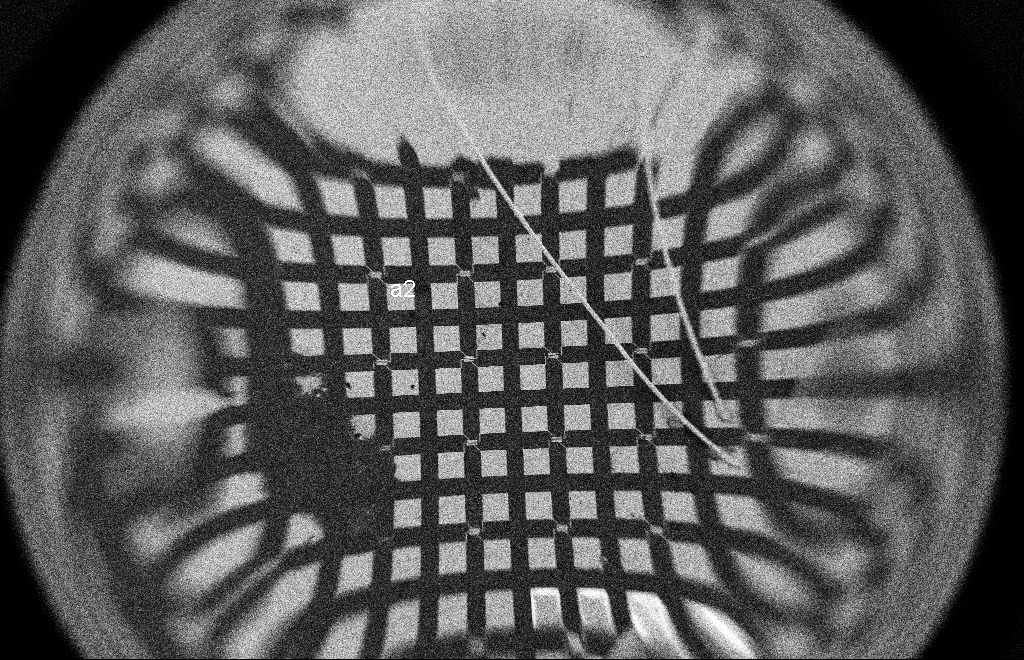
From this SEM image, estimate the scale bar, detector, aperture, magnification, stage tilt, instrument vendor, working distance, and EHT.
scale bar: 200000 nm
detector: SE2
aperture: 20 µm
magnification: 0.061 K X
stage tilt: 0°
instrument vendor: Zeiss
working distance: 9 mm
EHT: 2 kV